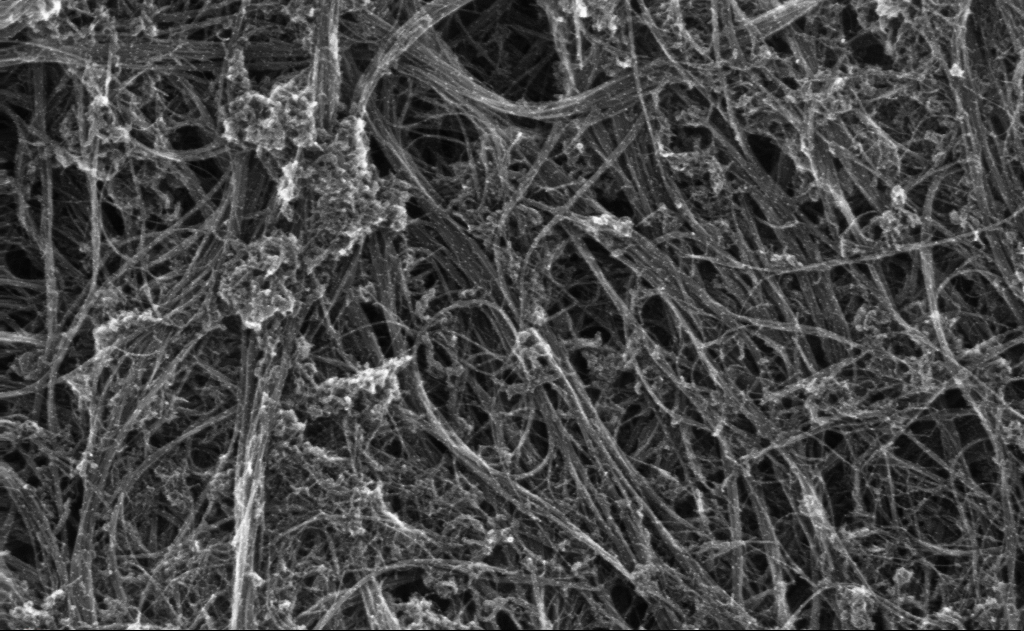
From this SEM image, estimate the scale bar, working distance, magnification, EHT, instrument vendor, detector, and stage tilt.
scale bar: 100 nm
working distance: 3 mm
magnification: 173.01 K X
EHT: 10 kV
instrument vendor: Zeiss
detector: InLens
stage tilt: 0°